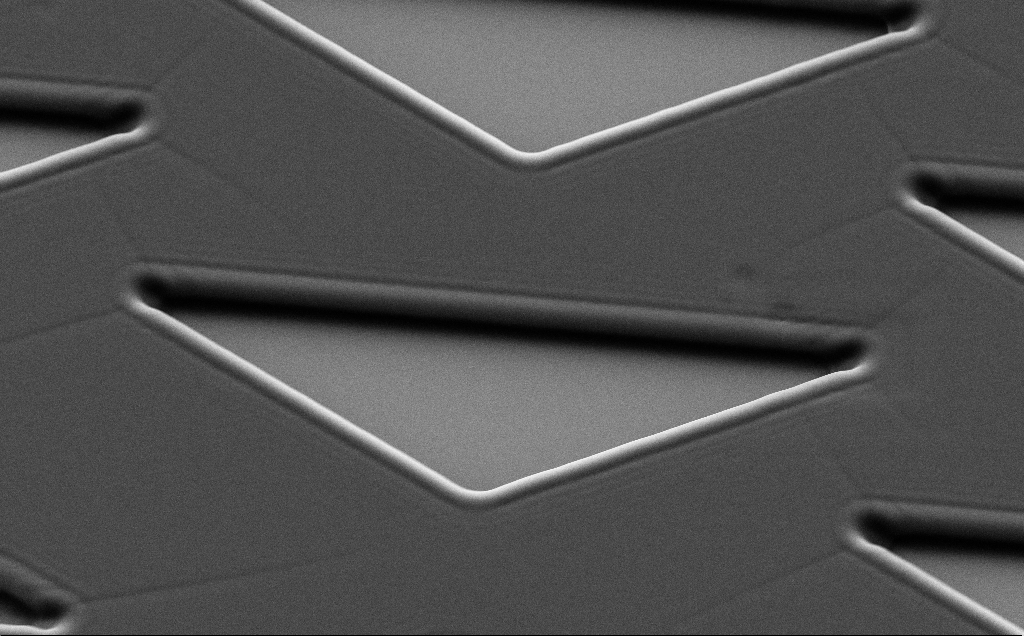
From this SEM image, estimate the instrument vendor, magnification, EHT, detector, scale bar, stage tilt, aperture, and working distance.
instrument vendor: Zeiss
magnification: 6.58 K X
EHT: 7 kV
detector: SE2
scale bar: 10000 nm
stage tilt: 35°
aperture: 30 µm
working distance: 5 mm